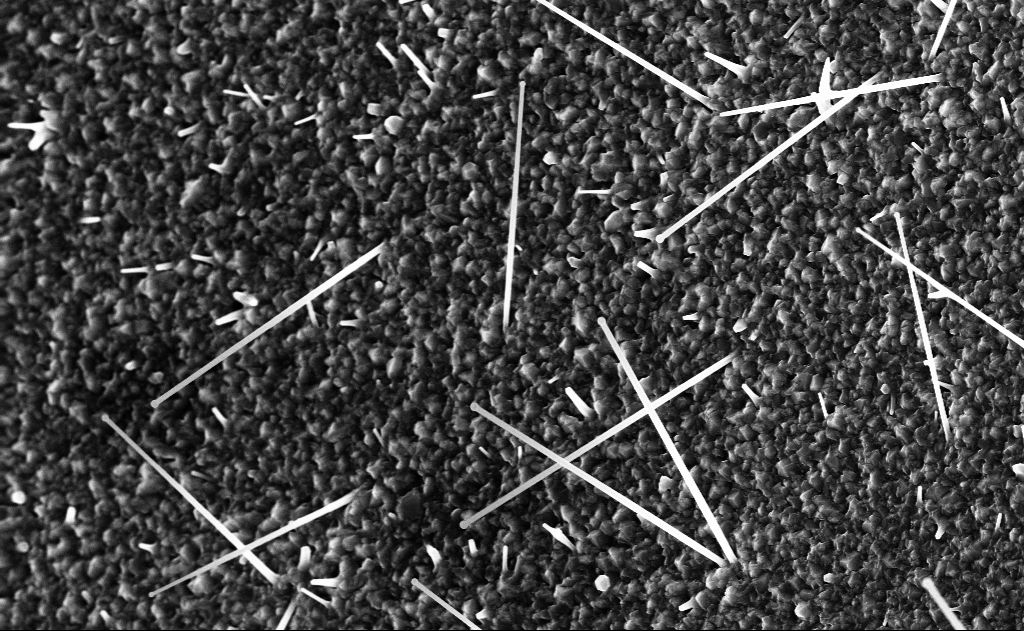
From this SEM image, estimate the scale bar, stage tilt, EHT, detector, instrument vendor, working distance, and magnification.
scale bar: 1000 nm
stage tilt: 0°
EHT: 10 kV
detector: InLens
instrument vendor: Zeiss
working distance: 9 mm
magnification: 20 K X